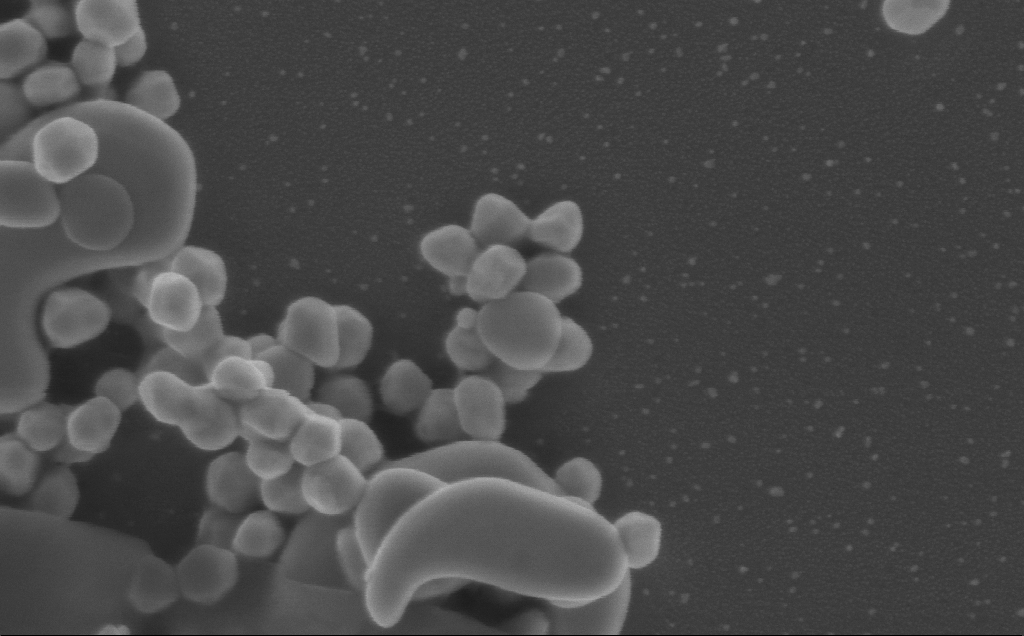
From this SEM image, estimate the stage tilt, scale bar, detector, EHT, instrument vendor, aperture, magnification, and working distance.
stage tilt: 0°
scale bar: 100 nm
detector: InLens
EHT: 5 kV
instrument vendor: Zeiss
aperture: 30 µm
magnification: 150 K X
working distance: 3 mm